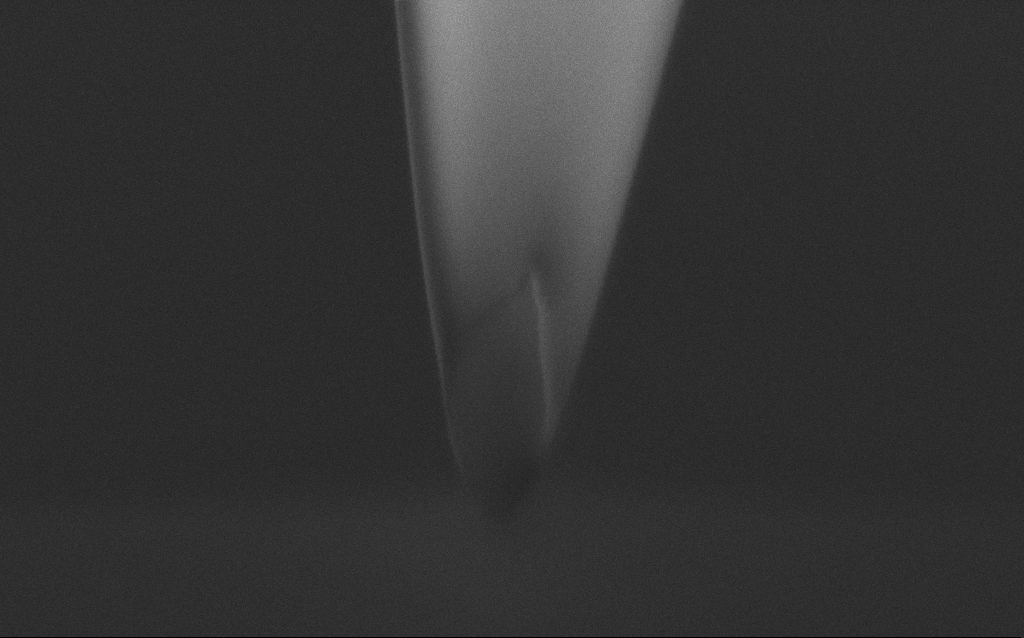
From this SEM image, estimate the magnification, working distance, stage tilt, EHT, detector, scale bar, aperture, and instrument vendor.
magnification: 155.81 K X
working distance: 5 mm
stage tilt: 45°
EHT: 2 kV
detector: InLens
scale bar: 200 nm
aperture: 30 µm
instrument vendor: Zeiss